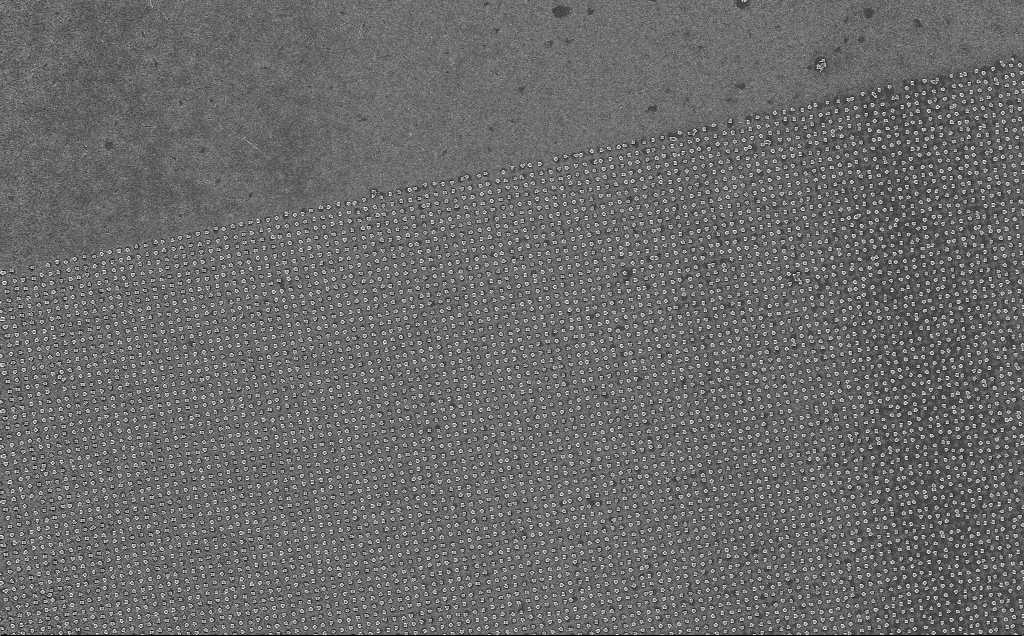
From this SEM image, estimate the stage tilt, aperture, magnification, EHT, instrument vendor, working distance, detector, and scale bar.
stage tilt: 0°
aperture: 30 µm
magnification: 2.2 K X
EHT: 5 kV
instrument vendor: Zeiss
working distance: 7 mm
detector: InLens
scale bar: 10000 nm